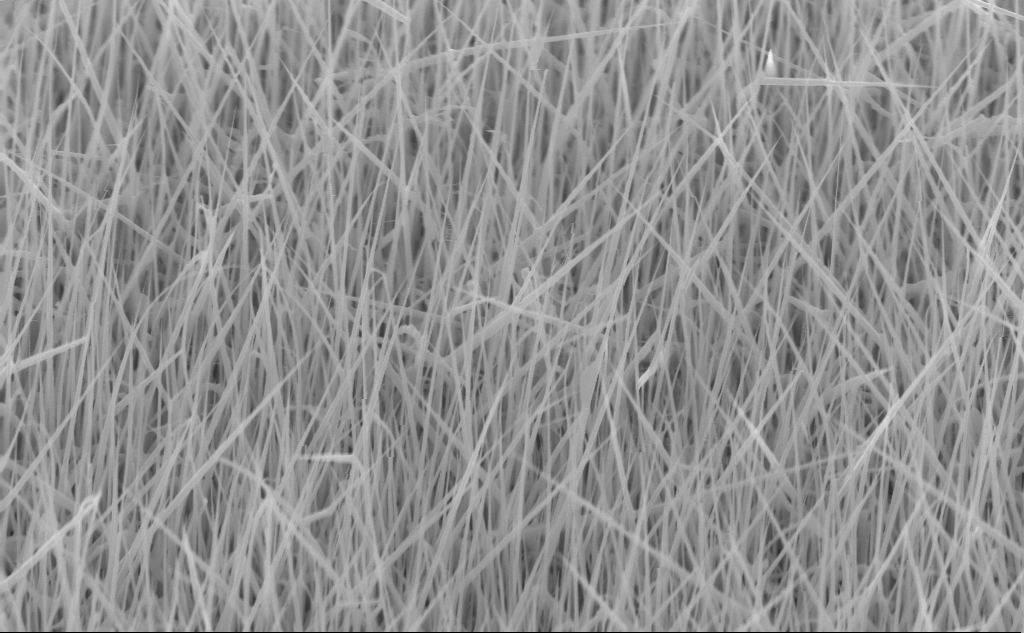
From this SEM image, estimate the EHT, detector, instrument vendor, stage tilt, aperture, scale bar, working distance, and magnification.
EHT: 10 kV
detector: InLens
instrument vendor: Zeiss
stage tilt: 45°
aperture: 30 µm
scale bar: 2000 nm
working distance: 4 mm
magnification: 30.43 K X